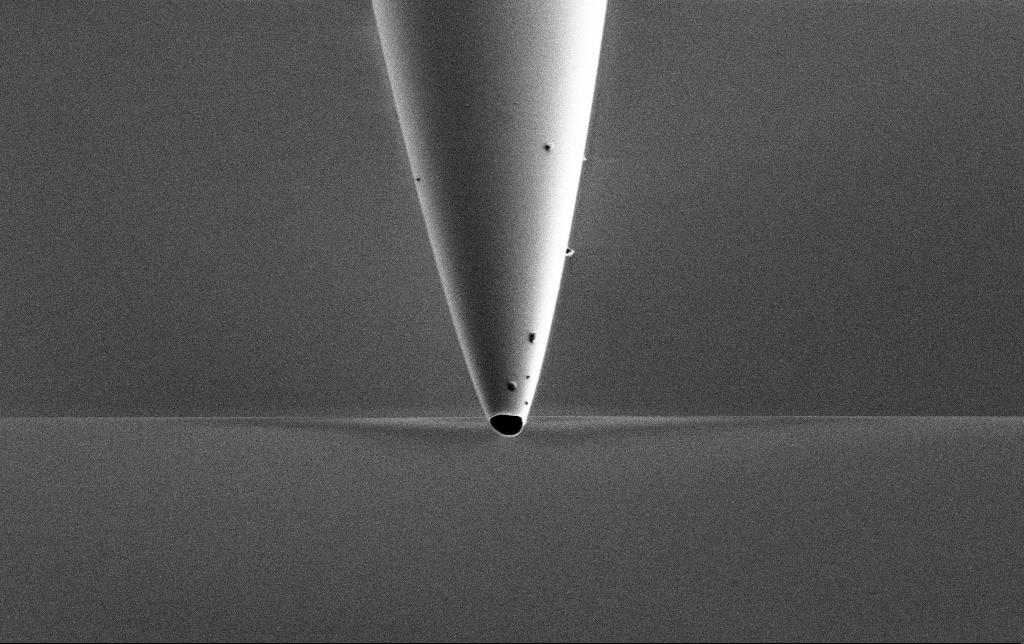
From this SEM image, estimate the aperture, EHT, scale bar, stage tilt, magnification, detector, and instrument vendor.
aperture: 30 µm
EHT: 1 kV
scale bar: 2000 nm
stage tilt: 45°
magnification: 15 K X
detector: SE2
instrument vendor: Zeiss